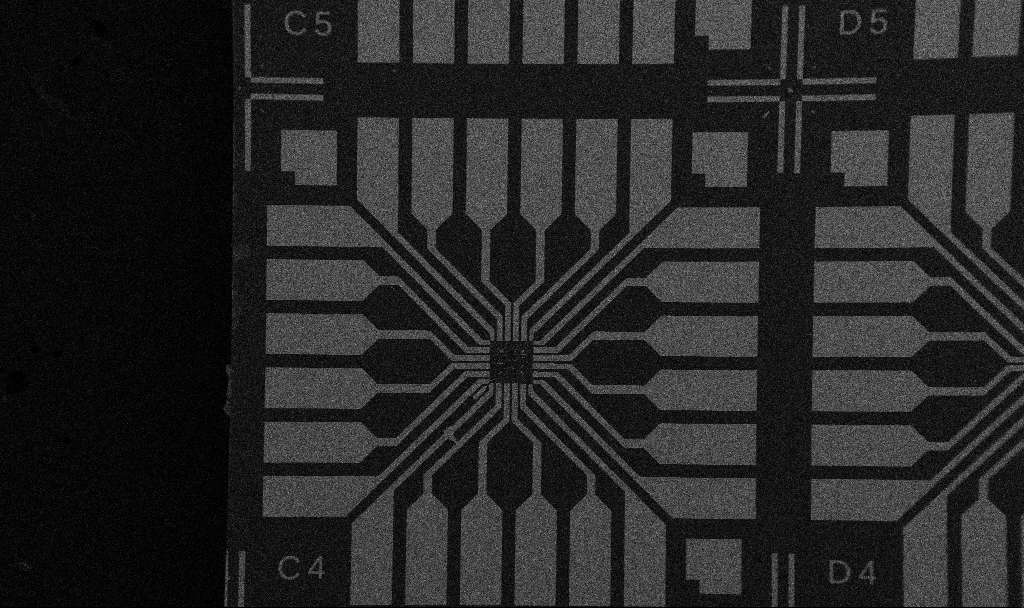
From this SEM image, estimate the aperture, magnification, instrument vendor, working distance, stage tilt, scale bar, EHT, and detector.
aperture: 30 µm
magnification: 0.1 K X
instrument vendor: Zeiss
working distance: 10.7 mm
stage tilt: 0°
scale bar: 200000 nm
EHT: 5 kV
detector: SE2